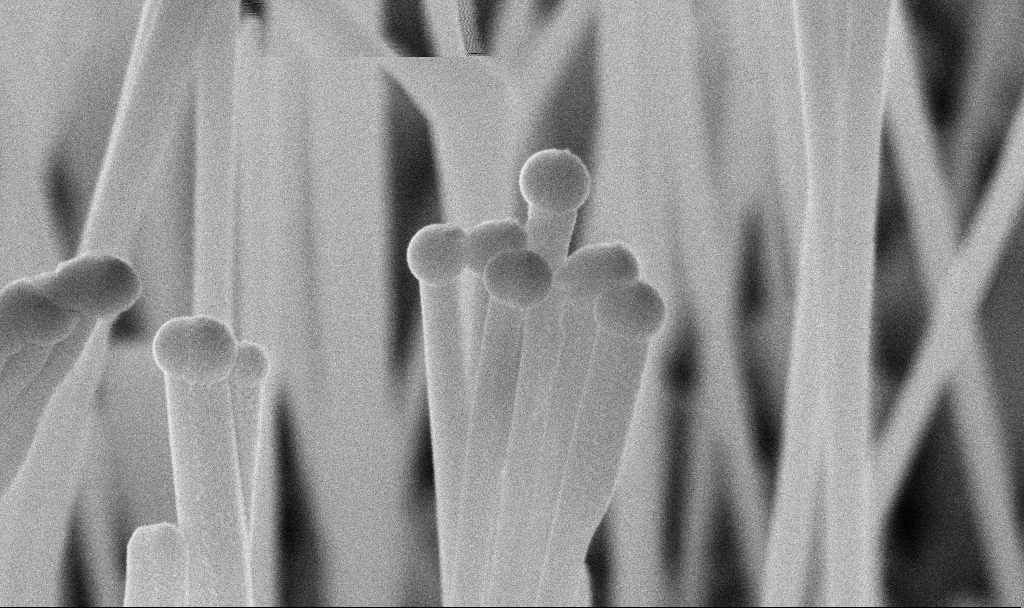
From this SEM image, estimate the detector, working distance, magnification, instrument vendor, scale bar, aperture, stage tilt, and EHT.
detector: InLens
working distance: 7 mm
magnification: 125.27 K X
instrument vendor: Zeiss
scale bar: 200 nm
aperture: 30 µm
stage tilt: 45°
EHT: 10 kV